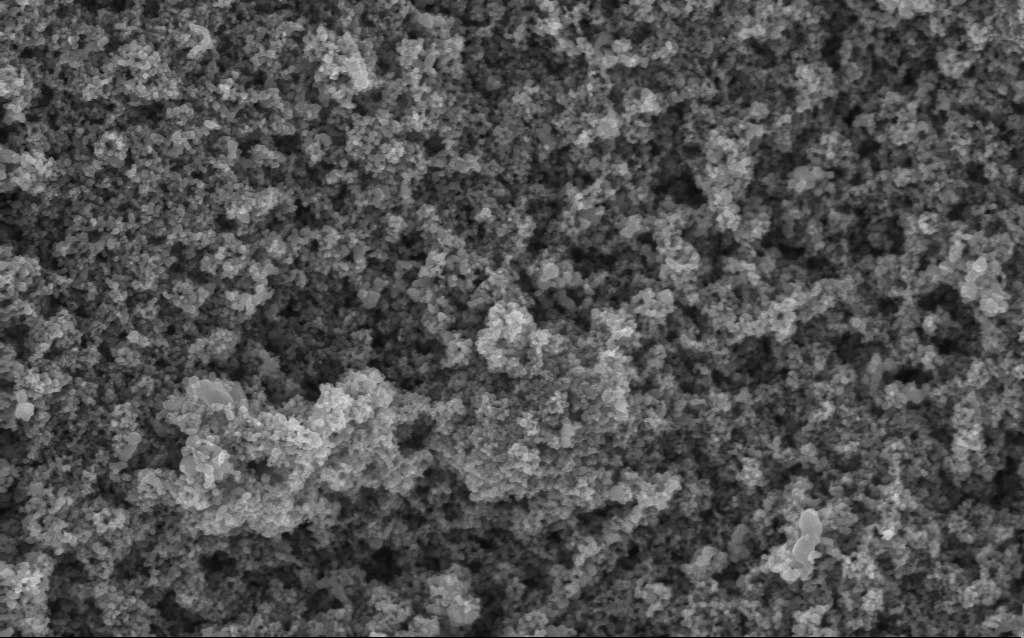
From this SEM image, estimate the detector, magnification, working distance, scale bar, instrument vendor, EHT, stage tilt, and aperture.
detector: InLens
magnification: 68.59 K X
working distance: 4.2 mm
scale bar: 1000 nm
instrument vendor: Zeiss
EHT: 5 kV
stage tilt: -0°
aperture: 30 µm